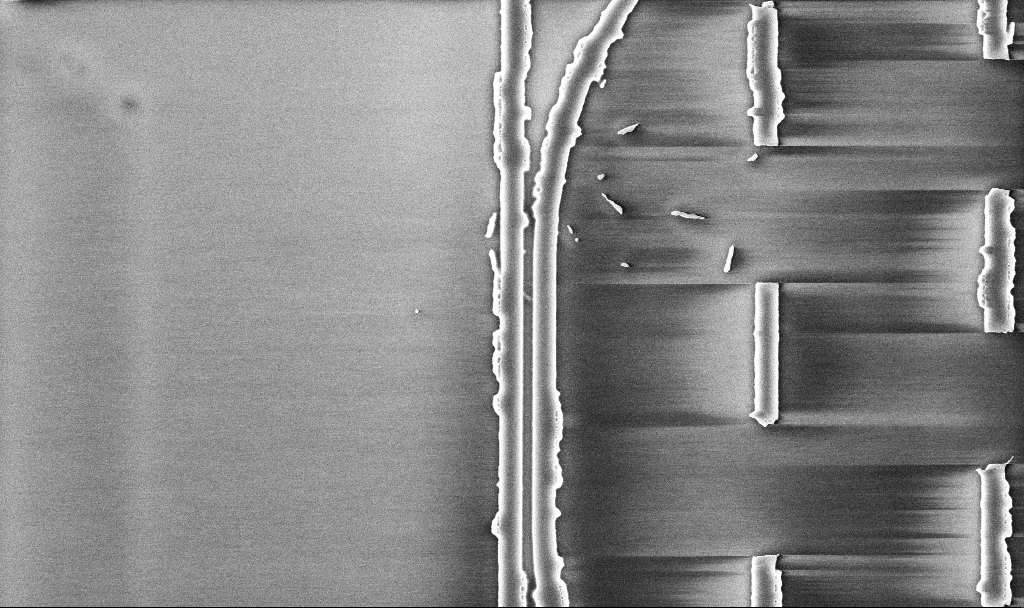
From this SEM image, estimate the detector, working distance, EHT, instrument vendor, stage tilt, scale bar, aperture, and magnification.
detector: InLens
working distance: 10.1 mm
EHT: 5 kV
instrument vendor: Zeiss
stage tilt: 0°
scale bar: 2000 nm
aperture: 30 µm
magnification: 17.08 K X